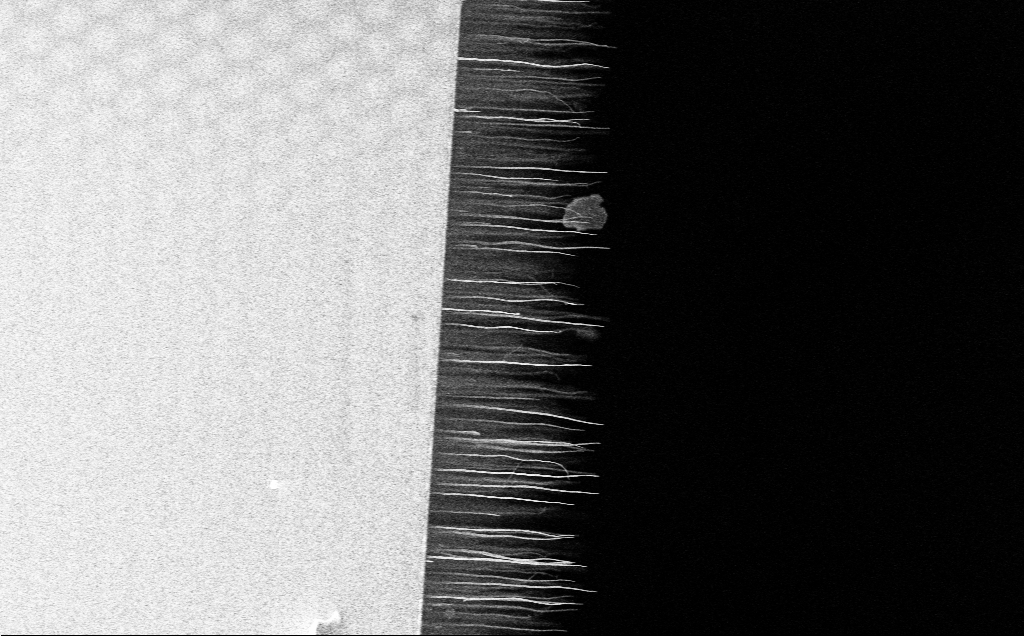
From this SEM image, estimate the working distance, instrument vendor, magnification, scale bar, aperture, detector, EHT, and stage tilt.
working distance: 12 mm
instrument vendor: Zeiss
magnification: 4.76 K X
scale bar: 10000 nm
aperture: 30 µm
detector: InLens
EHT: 5 kV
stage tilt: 0°